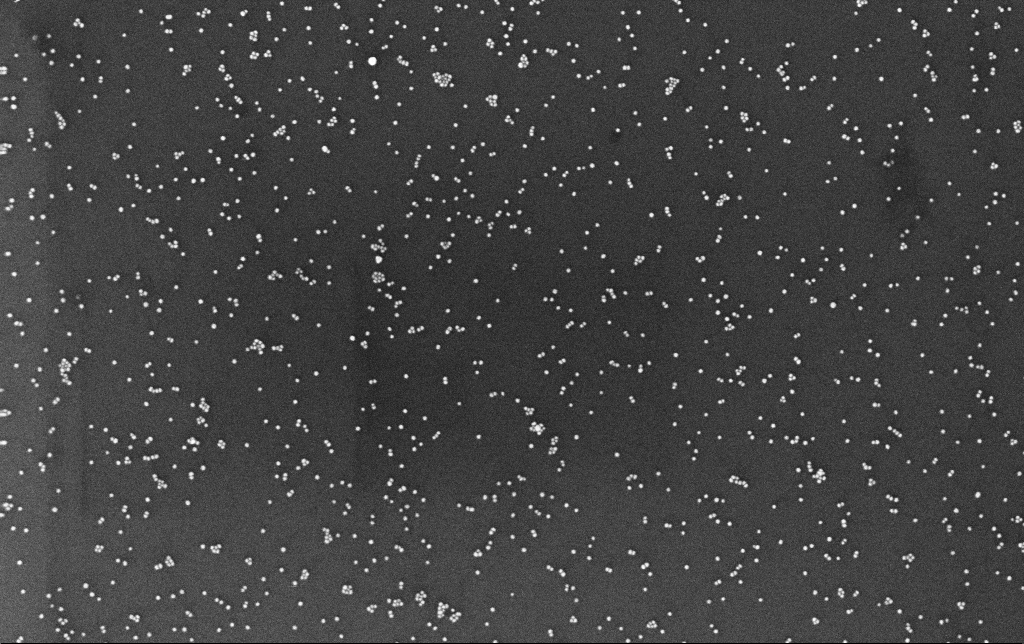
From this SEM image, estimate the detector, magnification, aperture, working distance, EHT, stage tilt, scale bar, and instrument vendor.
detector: InLens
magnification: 100 K X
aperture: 30 µm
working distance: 3.4 mm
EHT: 10 kV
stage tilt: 0°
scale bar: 200 nm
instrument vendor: Zeiss